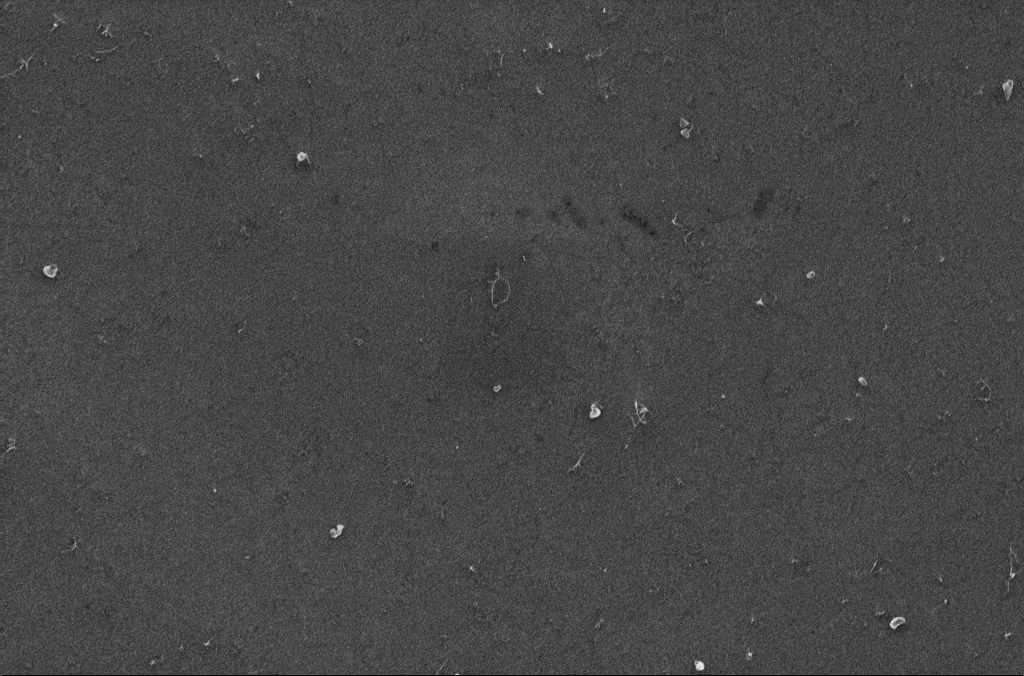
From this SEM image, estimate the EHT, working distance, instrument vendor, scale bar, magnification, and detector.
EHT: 10 kV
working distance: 3.4 mm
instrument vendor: Zeiss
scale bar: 1000 nm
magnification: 39.48 K X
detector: InLens